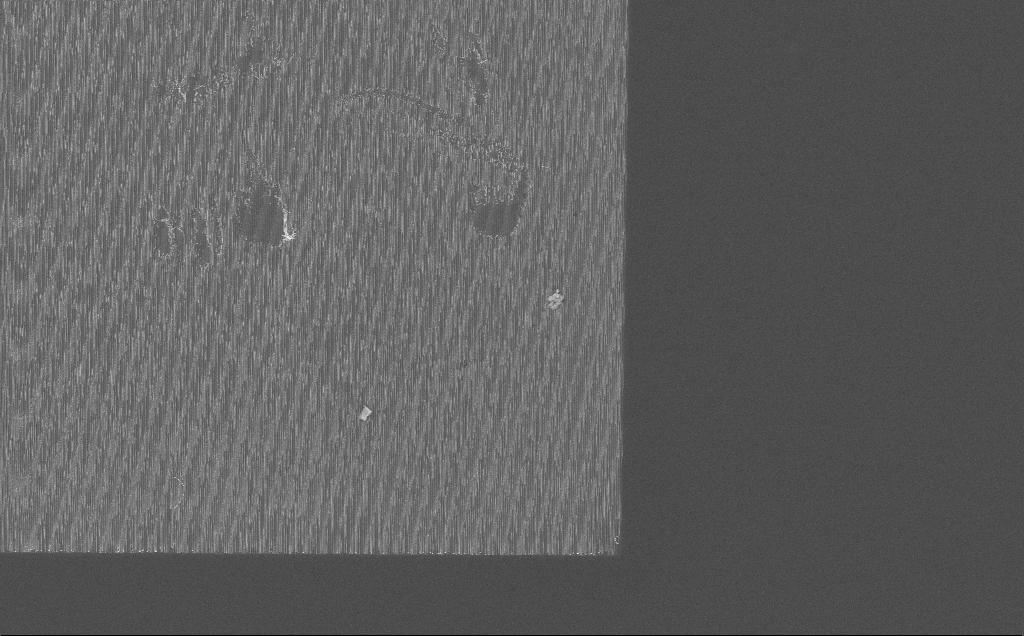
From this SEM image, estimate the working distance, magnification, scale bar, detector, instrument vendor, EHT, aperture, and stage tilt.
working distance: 7 mm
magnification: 2.77 K X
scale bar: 10000 nm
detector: InLens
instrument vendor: Zeiss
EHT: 10 kV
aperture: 30 µm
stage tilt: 0°